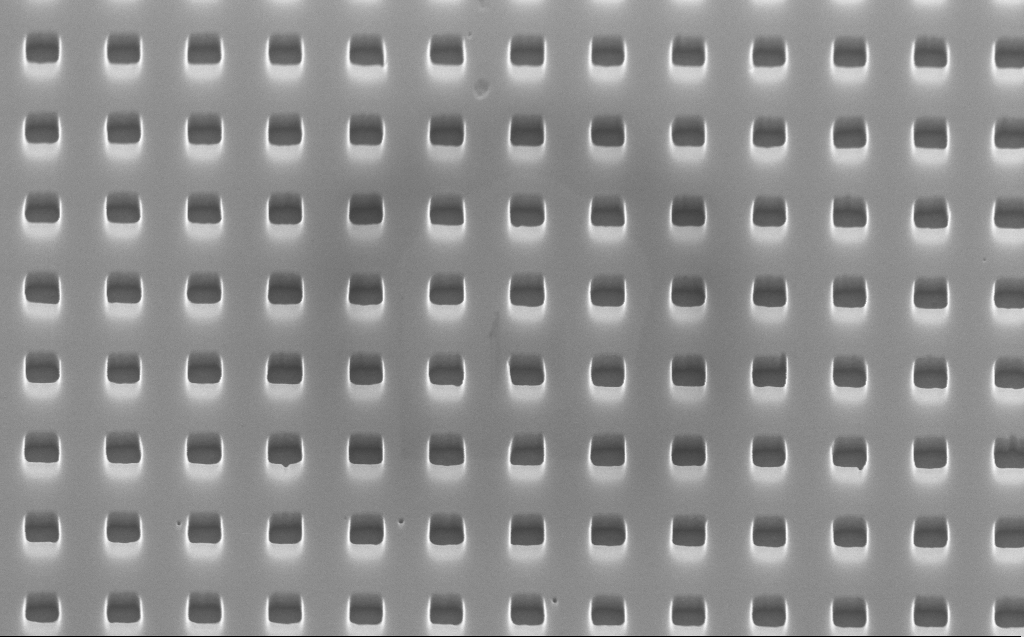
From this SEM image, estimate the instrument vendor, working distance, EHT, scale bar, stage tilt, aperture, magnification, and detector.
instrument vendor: Zeiss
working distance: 3 mm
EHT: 10 kV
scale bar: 1000 nm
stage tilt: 45°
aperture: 30 µm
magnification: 60 K X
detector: InLens